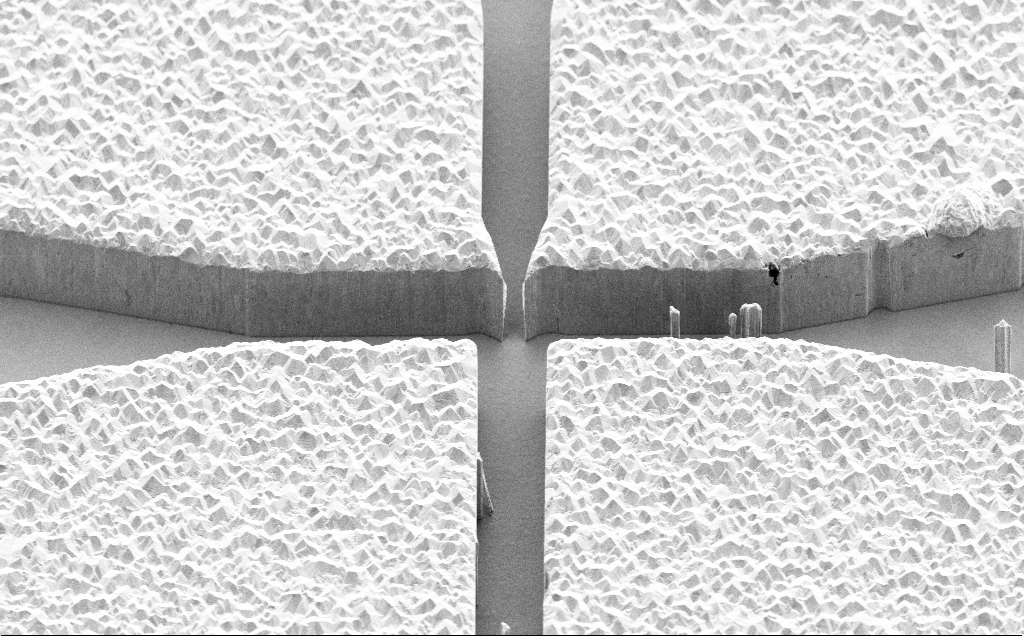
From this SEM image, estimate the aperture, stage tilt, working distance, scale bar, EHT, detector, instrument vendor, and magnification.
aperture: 30 µm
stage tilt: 45°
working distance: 10 mm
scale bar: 10000 nm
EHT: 5 kV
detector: SE2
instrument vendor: Zeiss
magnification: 2.49 K X